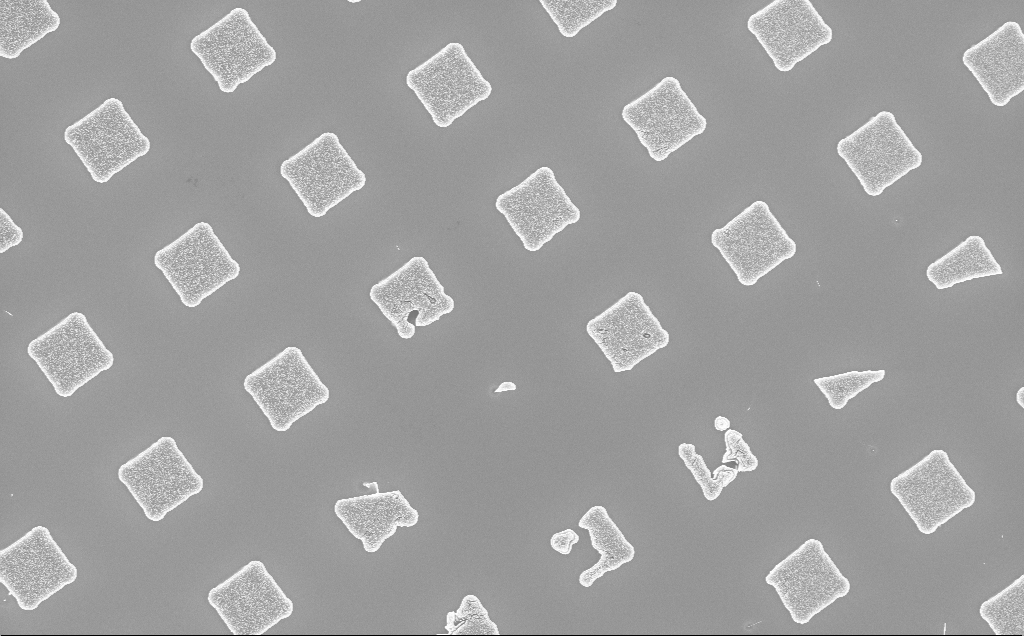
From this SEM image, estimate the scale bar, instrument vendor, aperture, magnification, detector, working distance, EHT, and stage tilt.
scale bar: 10000 nm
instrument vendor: Zeiss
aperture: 30 µm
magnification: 2.84 K X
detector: InLens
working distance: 12 mm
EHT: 10 kV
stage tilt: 0°